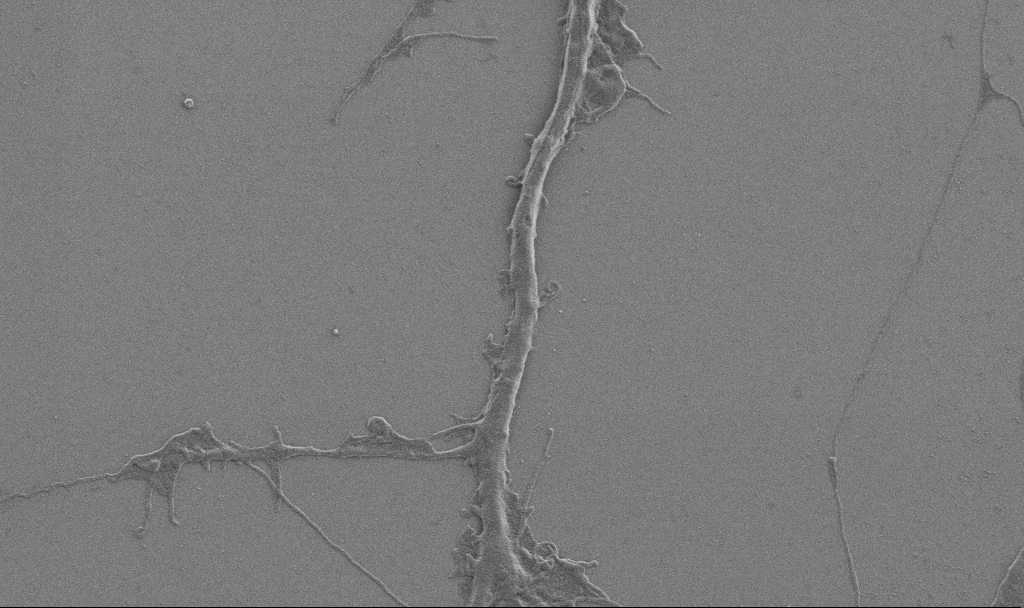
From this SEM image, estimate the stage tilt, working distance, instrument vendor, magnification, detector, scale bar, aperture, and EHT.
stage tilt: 0°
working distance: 6.9 mm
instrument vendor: Zeiss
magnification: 10 K X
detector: SE2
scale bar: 2000 nm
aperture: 30 µm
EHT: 0.9 kV